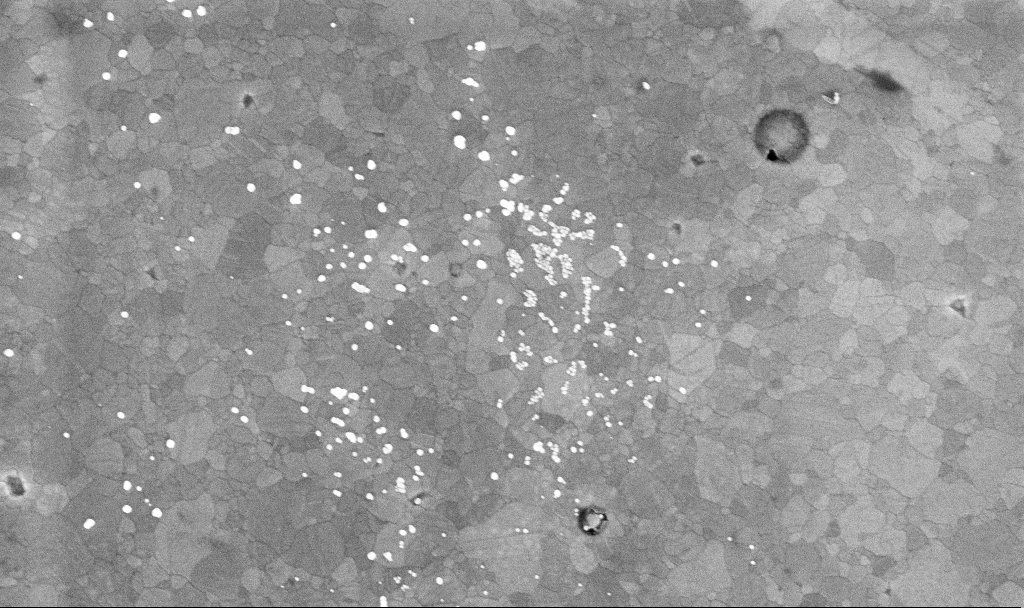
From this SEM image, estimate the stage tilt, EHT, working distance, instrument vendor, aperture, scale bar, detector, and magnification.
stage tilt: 0°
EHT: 10 kV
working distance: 3.4 mm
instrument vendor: Zeiss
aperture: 30 µm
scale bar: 1000 nm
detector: InLens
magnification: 52.02 K X